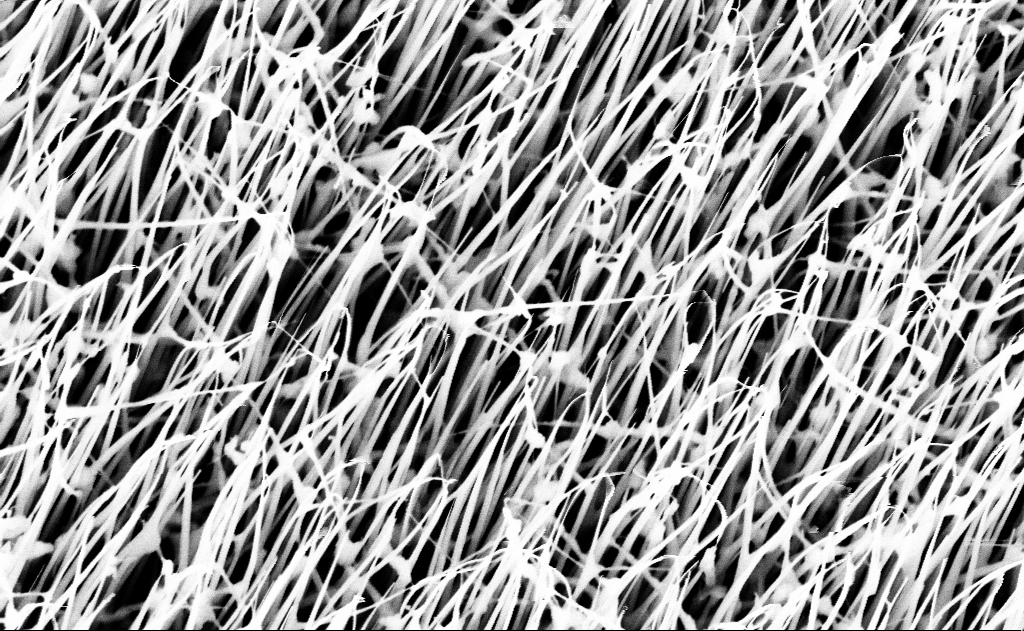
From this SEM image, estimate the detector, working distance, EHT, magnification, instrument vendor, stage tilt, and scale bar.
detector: InLens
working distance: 13 mm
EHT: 10 kV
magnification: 40 K X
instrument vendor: Zeiss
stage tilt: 0°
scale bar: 1000 nm